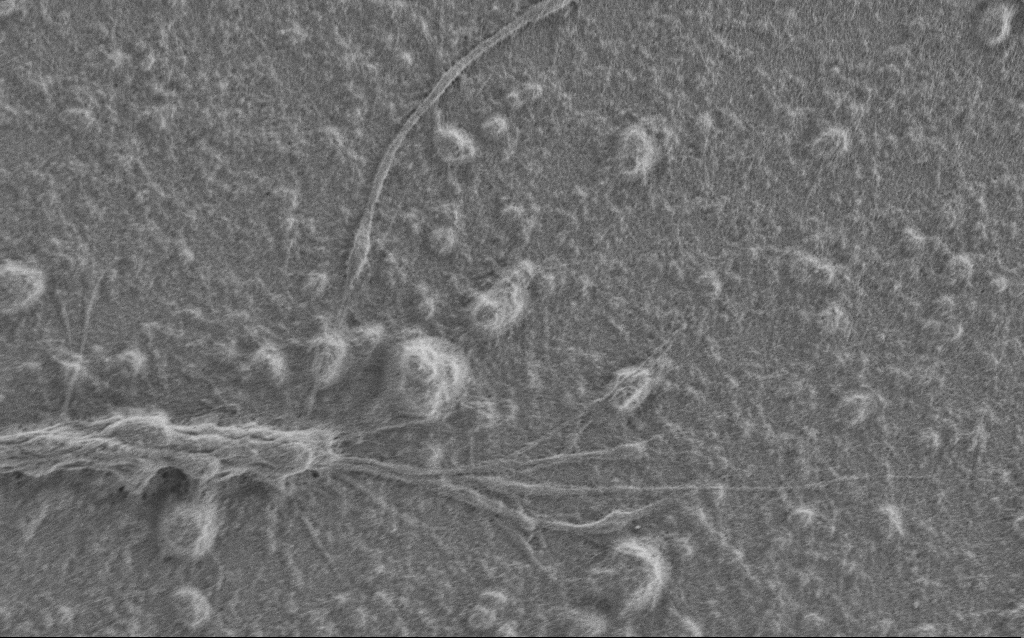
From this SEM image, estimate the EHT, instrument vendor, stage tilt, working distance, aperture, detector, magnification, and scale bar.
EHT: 1 kV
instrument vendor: Zeiss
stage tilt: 0°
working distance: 6 mm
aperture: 30 µm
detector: SE2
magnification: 7.5 K X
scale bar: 2000 nm